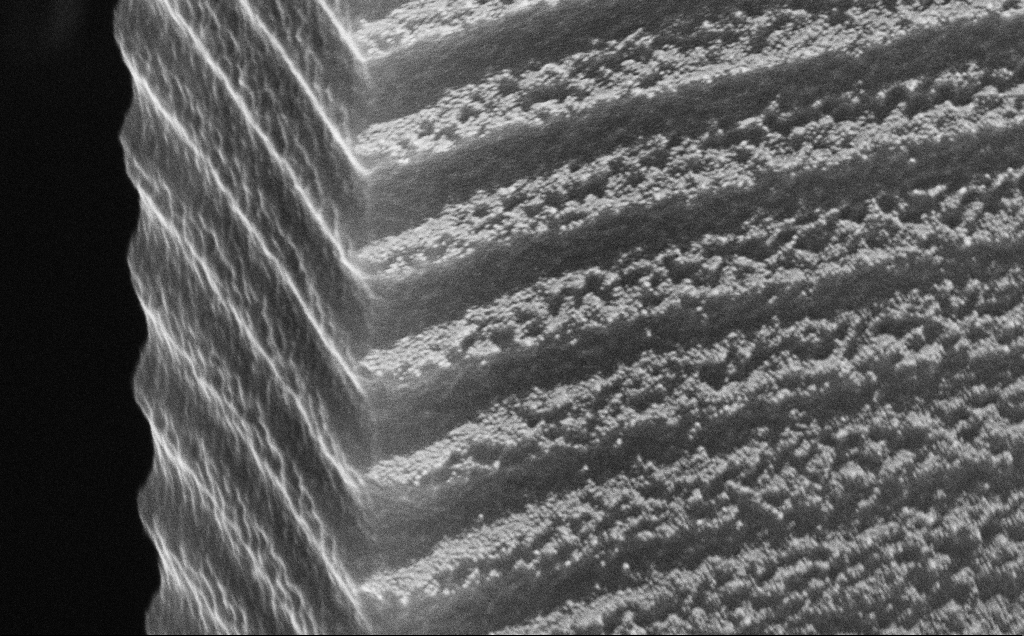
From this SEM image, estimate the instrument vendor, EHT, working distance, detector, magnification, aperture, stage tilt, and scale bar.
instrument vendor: Zeiss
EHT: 10 kV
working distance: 5 mm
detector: InLens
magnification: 109.84 K X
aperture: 30 µm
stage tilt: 60°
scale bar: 200 nm